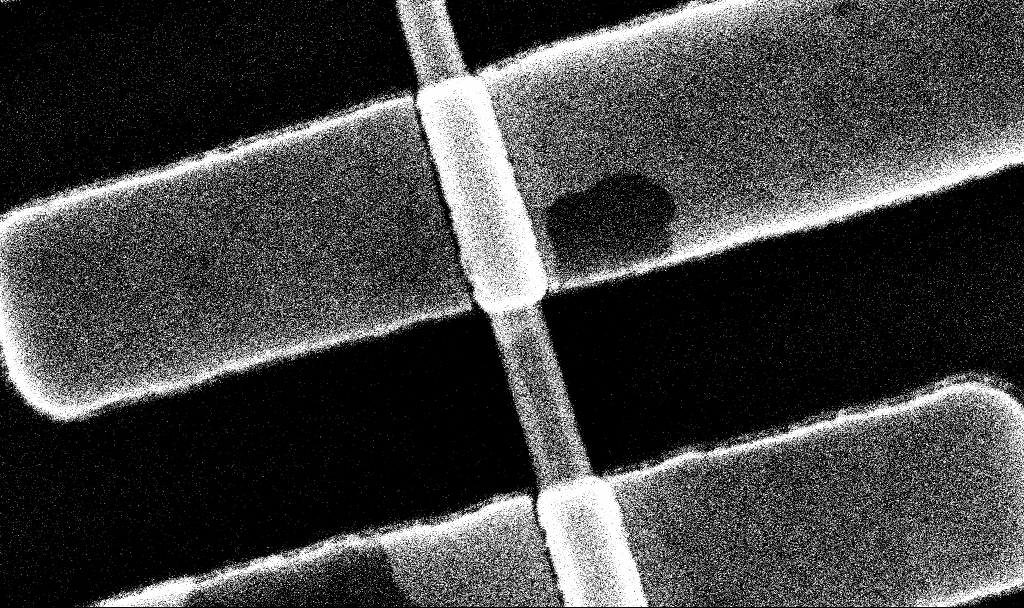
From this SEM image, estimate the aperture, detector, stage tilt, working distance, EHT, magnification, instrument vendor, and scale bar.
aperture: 30 µm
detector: InLens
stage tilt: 0°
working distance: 6.7 mm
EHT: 10 kV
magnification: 136.1 K X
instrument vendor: Zeiss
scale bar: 200 nm